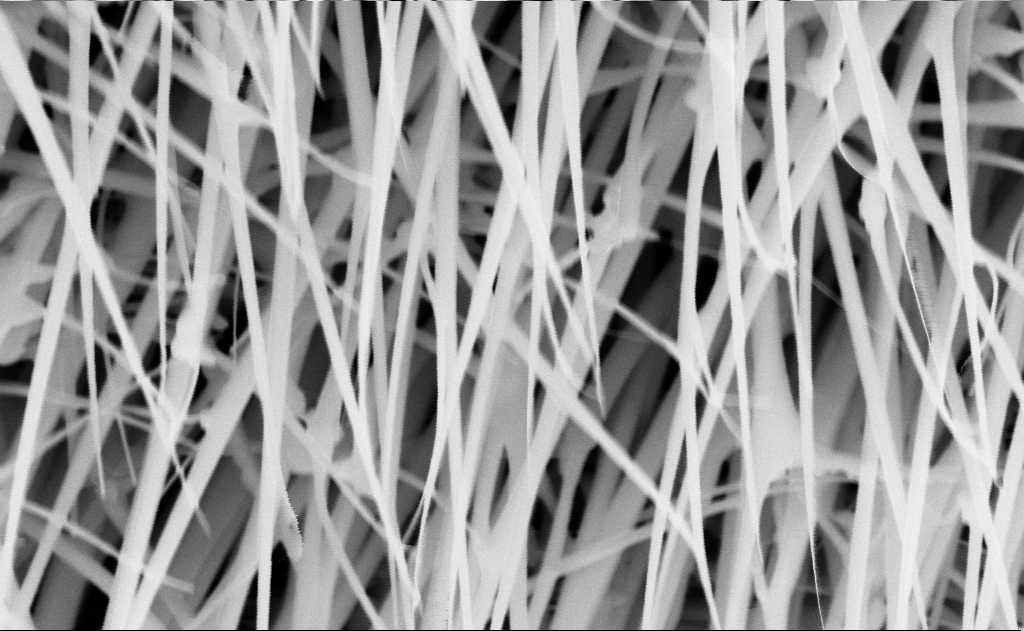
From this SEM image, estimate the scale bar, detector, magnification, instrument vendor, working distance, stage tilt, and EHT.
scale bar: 200 nm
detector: InLens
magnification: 80 K X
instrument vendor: Zeiss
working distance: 13 mm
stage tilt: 0°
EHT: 10 kV